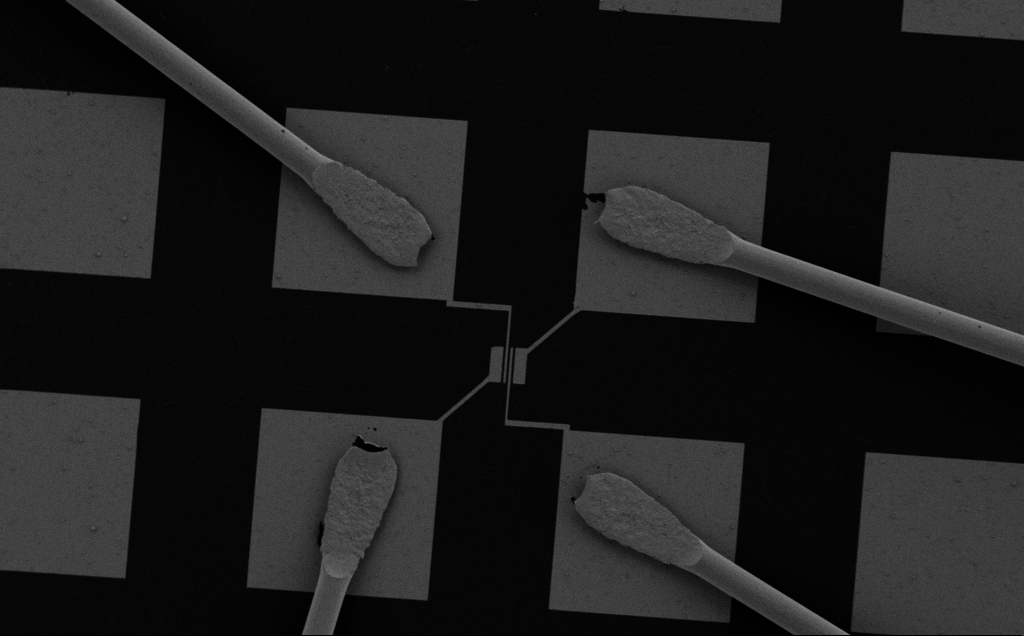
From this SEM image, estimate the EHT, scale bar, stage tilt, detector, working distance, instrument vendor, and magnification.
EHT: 5 kV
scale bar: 100000 nm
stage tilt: -0.7°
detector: SE2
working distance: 8 mm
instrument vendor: Zeiss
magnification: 0.449 K X